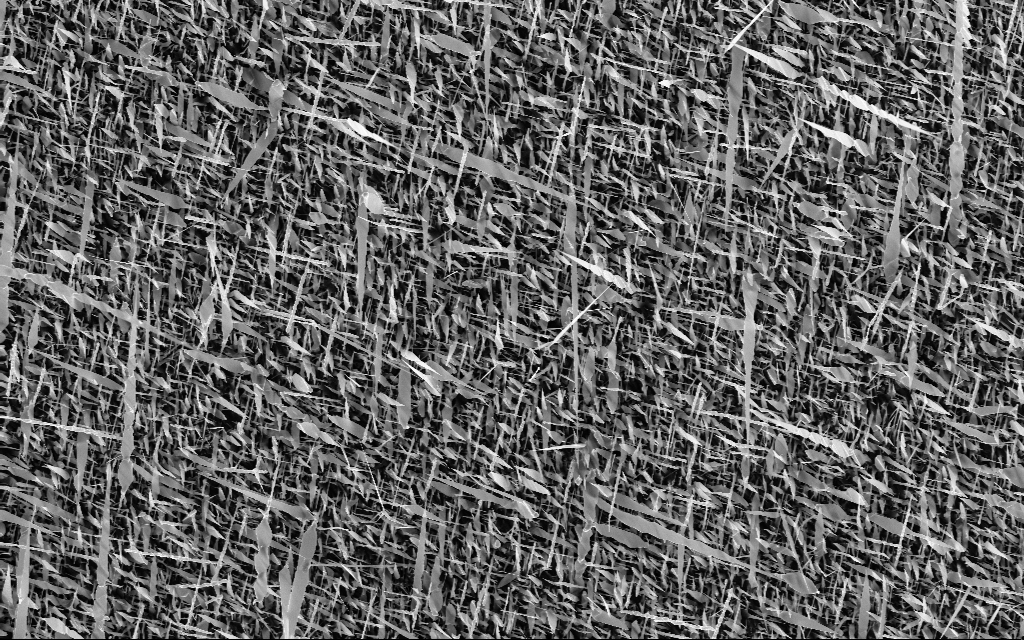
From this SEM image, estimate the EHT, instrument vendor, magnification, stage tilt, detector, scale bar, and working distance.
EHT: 10 kV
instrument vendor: Zeiss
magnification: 5 K X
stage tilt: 0°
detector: InLens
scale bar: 10000 nm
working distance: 6 mm